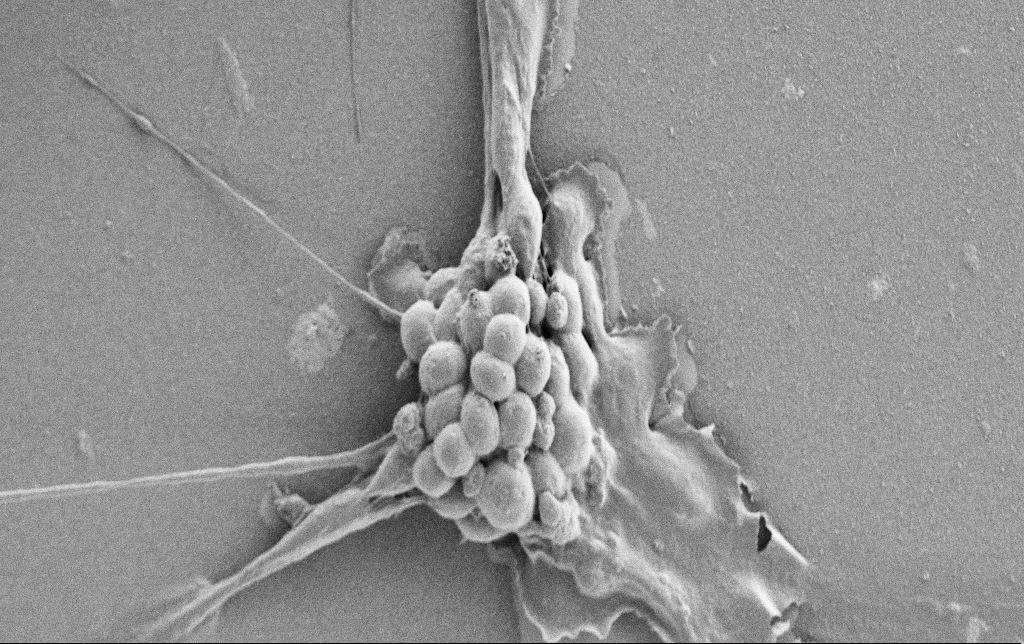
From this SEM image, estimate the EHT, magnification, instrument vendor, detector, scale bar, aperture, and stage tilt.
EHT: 0.9 kV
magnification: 3.5 K X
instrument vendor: Zeiss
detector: SE2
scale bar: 10000 nm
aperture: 30 µm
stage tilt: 0°